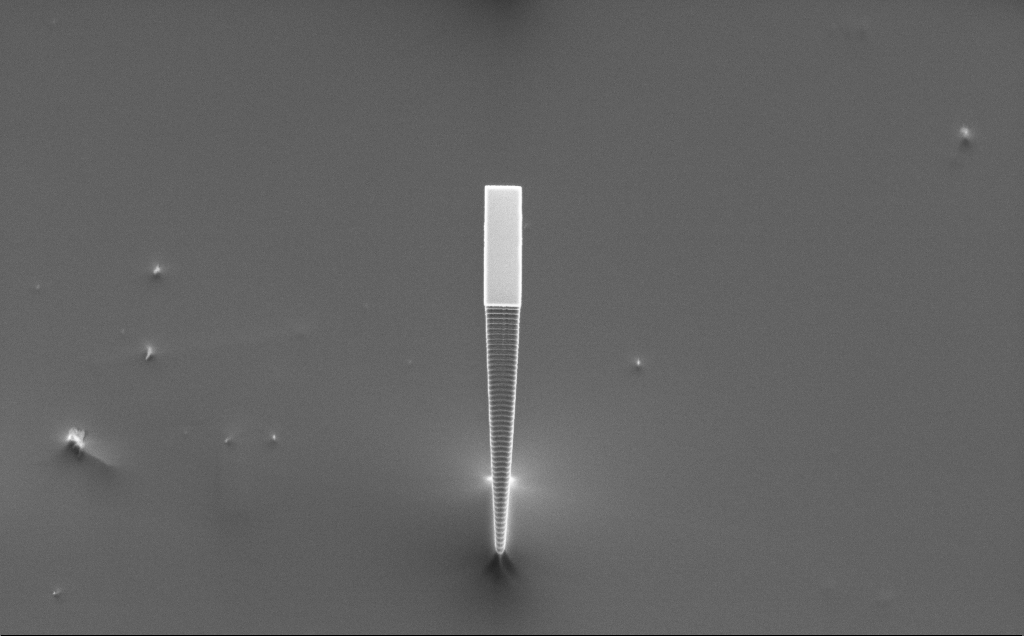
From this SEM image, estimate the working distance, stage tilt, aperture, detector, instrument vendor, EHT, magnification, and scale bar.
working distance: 16 mm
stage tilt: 50°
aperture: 30 µm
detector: SE2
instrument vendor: Zeiss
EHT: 5 kV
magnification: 4.47 K X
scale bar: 10000 nm